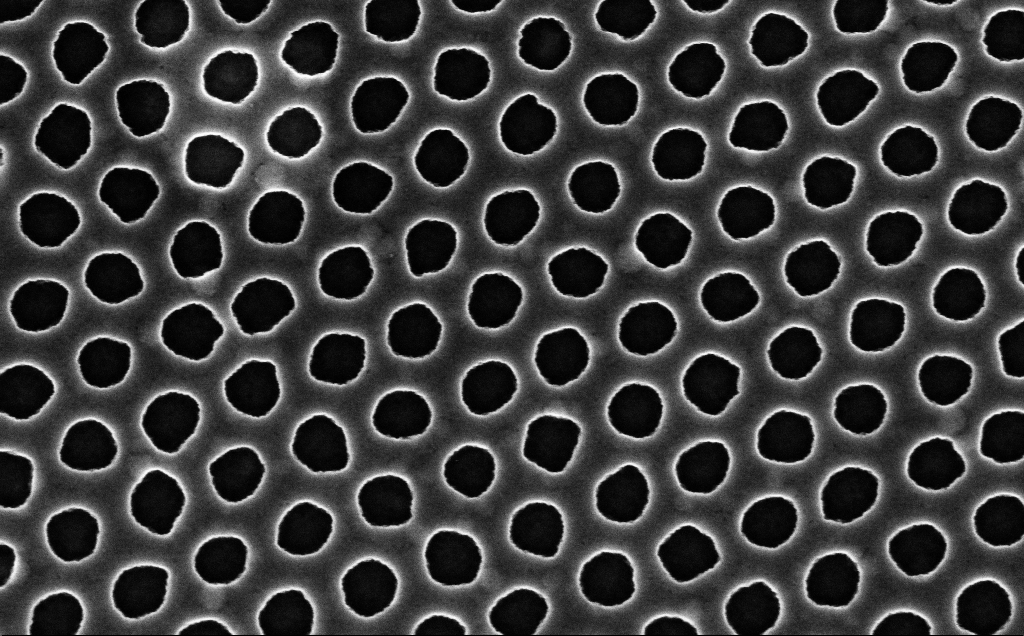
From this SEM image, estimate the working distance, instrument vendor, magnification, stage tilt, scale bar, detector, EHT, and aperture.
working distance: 2.5 mm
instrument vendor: Zeiss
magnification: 90 K X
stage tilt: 0°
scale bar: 200 nm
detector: InLens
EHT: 3 kV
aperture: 30 µm